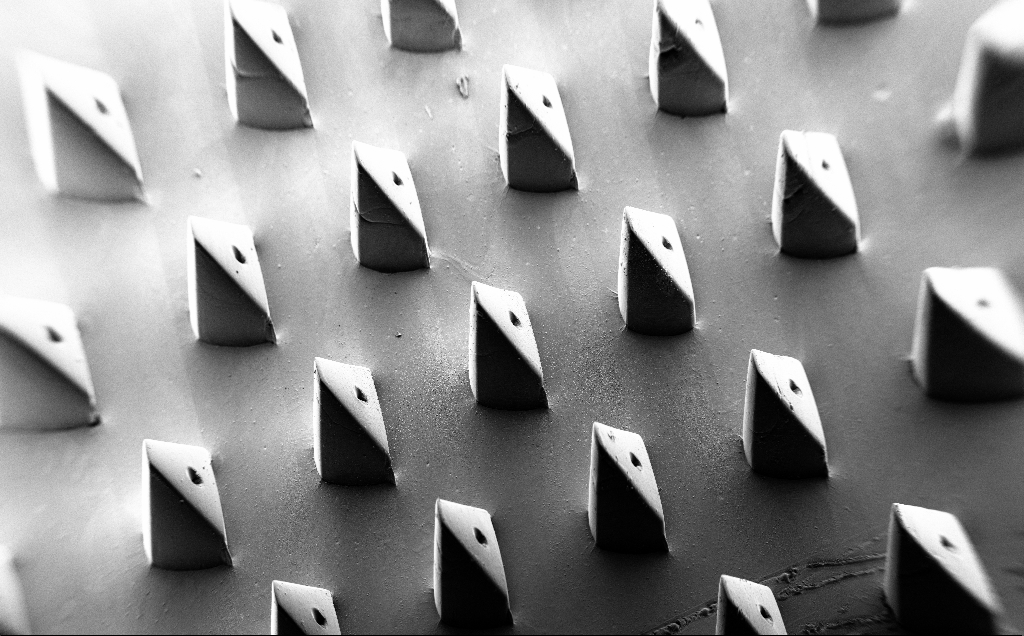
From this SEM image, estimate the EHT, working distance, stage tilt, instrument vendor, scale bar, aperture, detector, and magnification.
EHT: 5 kV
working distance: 9 mm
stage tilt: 35°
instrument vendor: Zeiss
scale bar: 1e+06 nm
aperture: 30 µm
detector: SE2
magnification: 0.067 K X